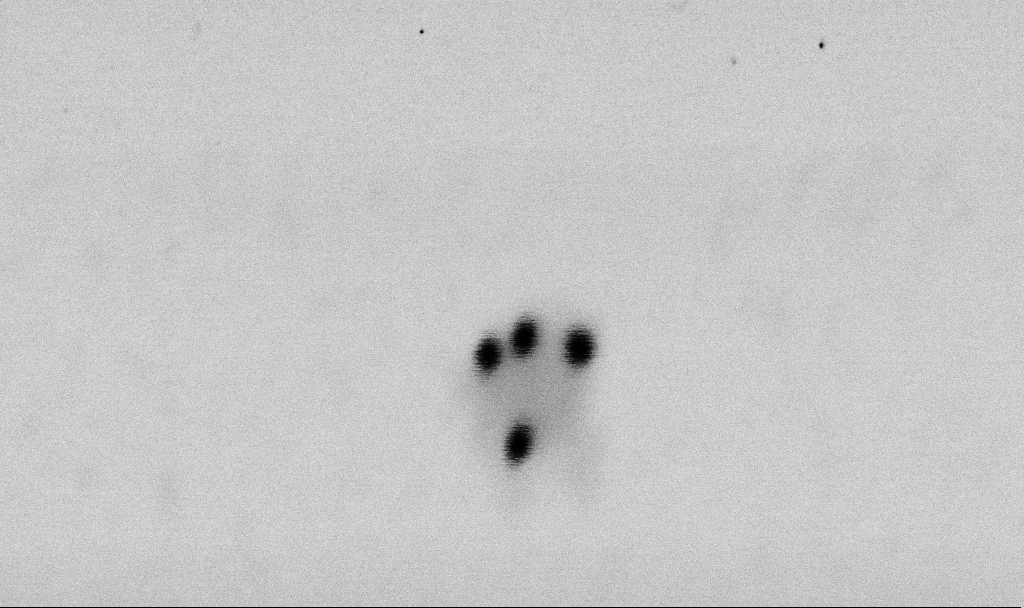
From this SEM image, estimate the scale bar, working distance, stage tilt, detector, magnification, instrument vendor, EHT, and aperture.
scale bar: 100 nm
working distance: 6.5 mm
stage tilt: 0°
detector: SE2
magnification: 400 K X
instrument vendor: Zeiss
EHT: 2 kV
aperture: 30 µm